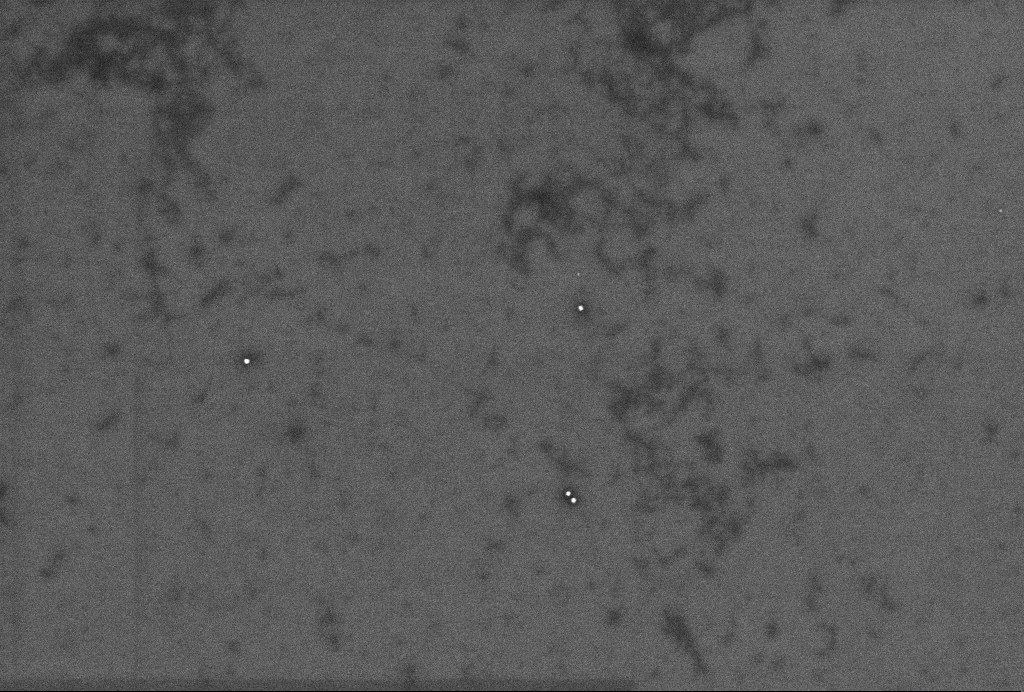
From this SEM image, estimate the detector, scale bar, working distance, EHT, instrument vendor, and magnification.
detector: InLens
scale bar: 200 nm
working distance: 3.3 mm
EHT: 2 kV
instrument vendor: Zeiss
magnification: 63.53 K X